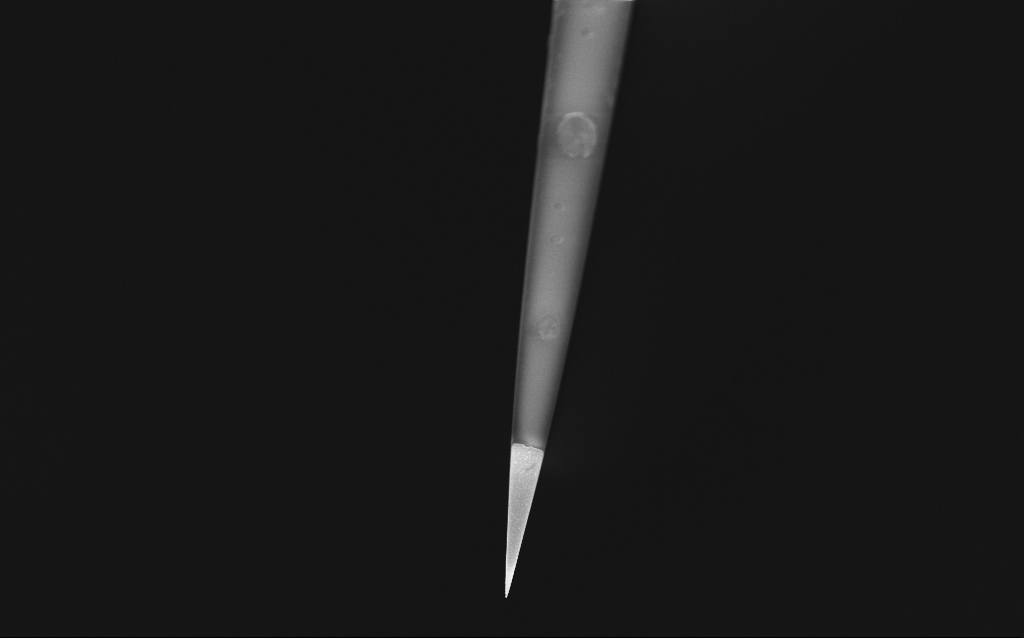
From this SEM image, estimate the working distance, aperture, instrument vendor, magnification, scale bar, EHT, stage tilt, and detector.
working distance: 6 mm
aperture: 30 µm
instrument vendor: Zeiss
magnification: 1 K X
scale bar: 20000 nm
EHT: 1 kV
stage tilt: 45°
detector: InLens